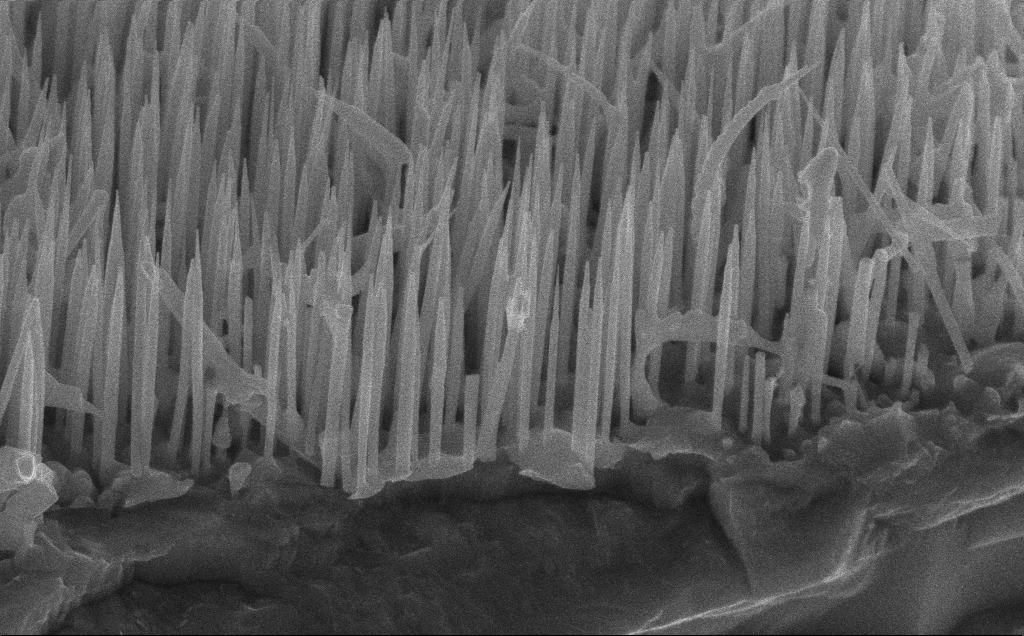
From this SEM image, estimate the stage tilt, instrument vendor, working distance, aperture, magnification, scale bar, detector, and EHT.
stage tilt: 45°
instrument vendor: Zeiss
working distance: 5 mm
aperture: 30 µm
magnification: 40 K X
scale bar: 1000 nm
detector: InLens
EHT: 12 kV